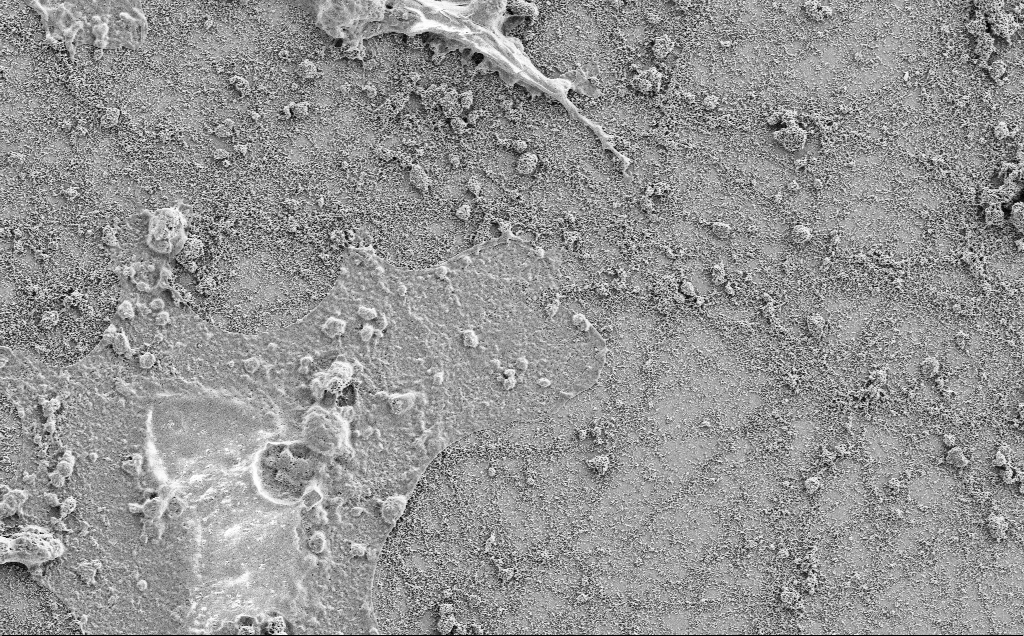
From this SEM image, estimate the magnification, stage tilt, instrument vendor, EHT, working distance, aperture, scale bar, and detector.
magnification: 3 K X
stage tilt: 0°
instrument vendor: Zeiss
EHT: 2 kV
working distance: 7.1 mm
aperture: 30 µm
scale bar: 10000 nm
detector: SE2